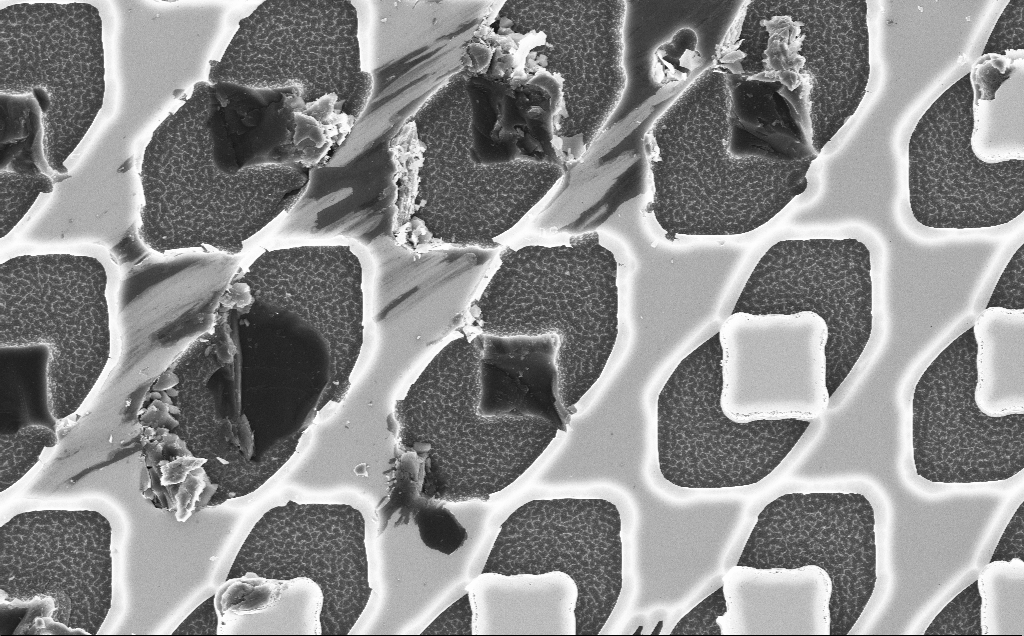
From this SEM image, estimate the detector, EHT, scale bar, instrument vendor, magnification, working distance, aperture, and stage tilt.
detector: InLens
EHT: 10 kV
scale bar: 10000 nm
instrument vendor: Zeiss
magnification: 4.69 K X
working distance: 9 mm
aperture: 30 µm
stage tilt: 0°